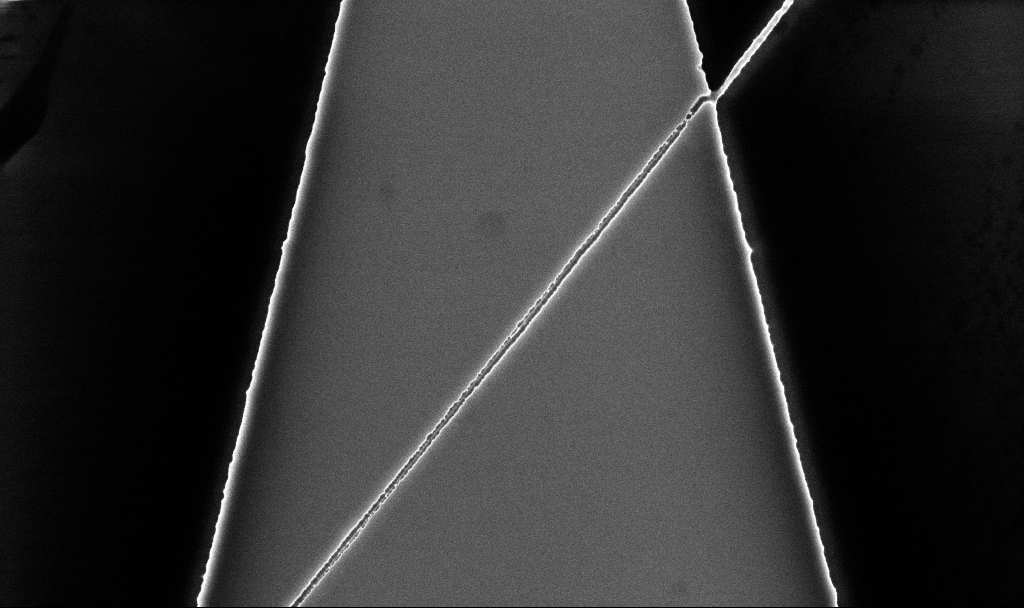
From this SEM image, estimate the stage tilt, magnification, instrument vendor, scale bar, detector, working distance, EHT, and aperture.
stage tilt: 0°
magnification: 19.15 K X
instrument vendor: Zeiss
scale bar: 1000 nm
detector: InLens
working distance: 5.2 mm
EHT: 5 kV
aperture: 30 µm